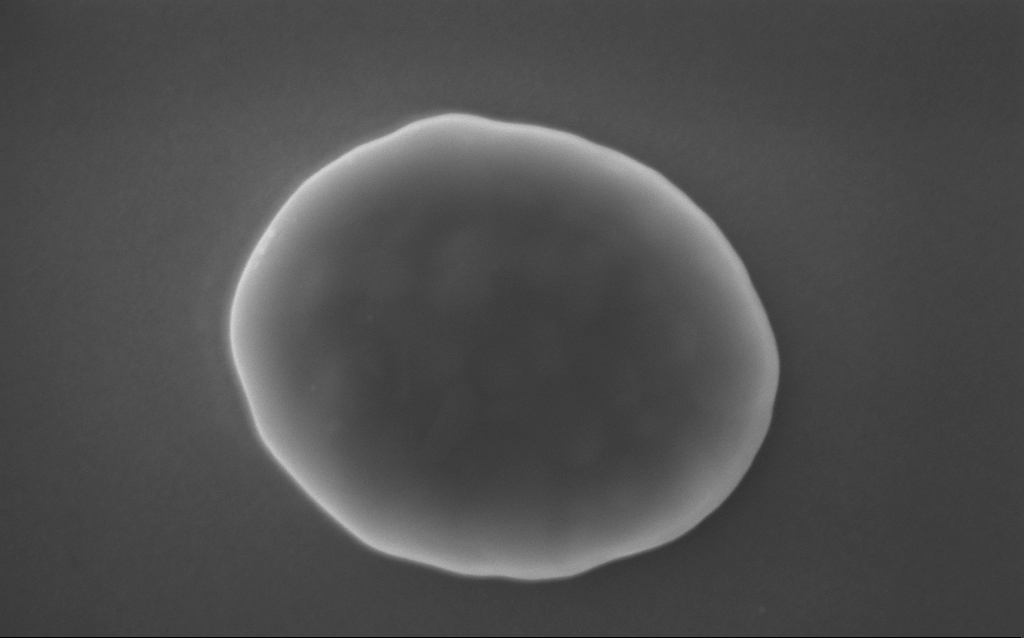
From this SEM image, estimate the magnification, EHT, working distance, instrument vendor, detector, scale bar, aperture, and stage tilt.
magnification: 195.04 K X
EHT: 10 kV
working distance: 3 mm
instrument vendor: Zeiss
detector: InLens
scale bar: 200 nm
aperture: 30 µm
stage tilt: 0°